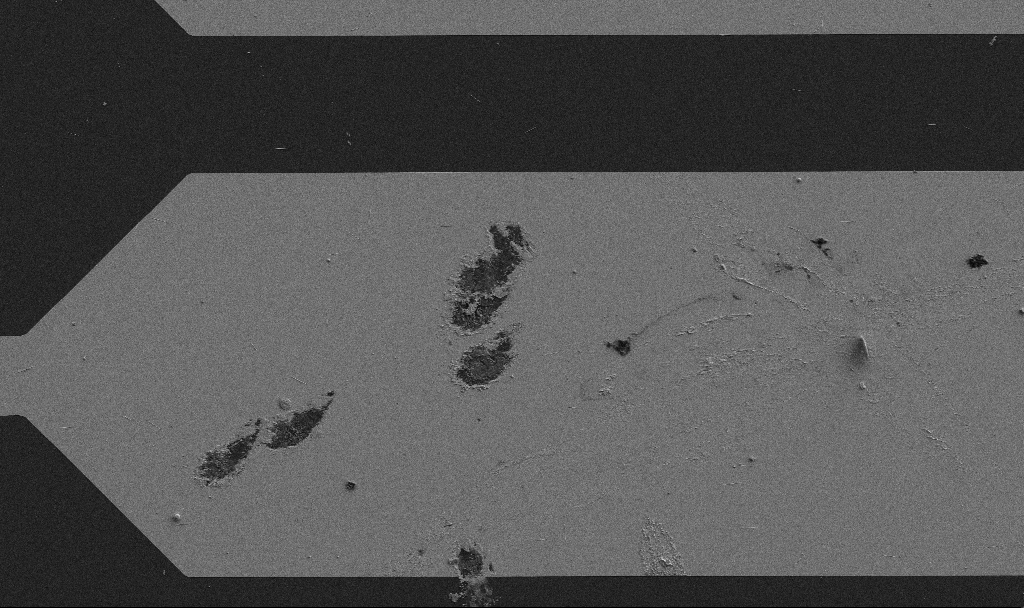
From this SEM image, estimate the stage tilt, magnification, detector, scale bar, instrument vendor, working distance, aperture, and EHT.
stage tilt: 0°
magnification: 1.01 K X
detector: SE2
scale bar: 20000 nm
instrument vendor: Zeiss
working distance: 8.5 mm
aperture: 30 µm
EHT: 5 kV